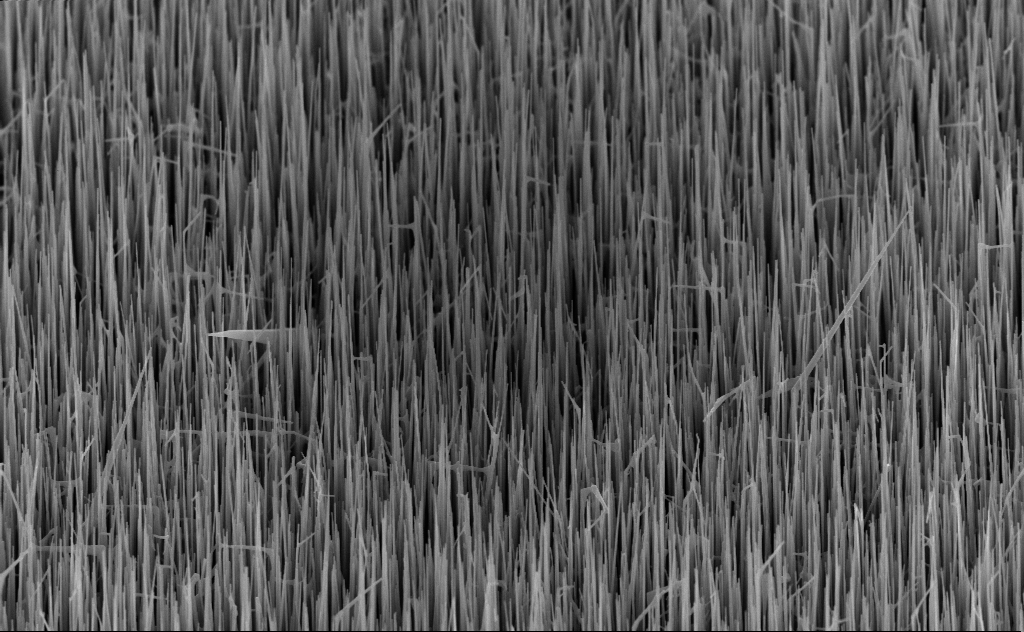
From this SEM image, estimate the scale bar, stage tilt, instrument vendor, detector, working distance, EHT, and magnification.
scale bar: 1000 nm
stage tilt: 45°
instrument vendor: Zeiss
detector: InLens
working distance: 6 mm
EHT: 10 kV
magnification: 20 K X